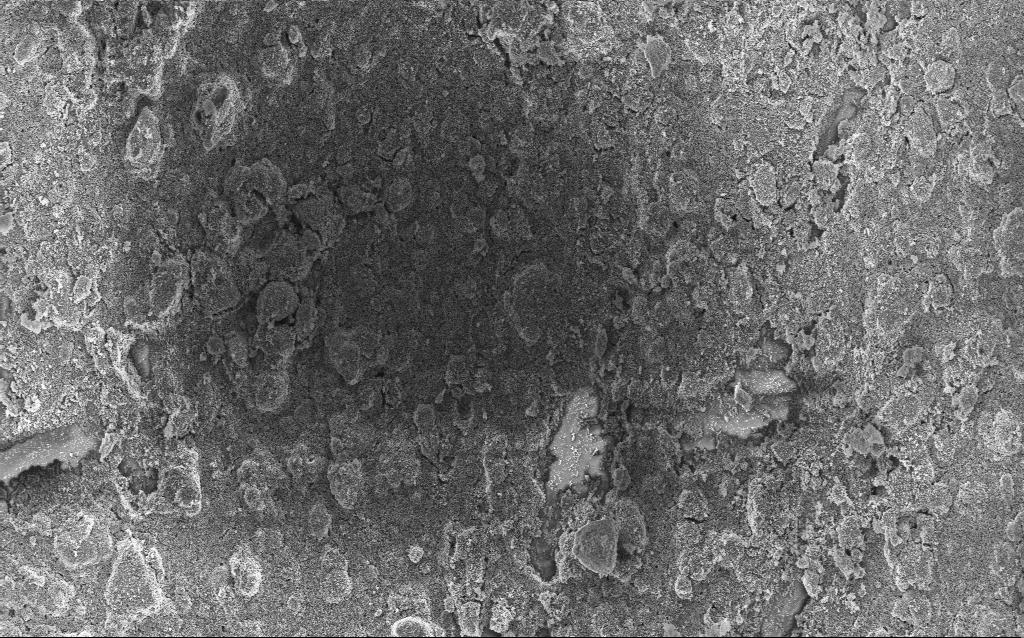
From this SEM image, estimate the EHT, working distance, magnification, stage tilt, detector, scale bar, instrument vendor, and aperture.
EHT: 5 kV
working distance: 2.5 mm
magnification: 1.69 K X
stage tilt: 0°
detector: InLens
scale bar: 10000 nm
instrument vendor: Zeiss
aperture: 30 µm